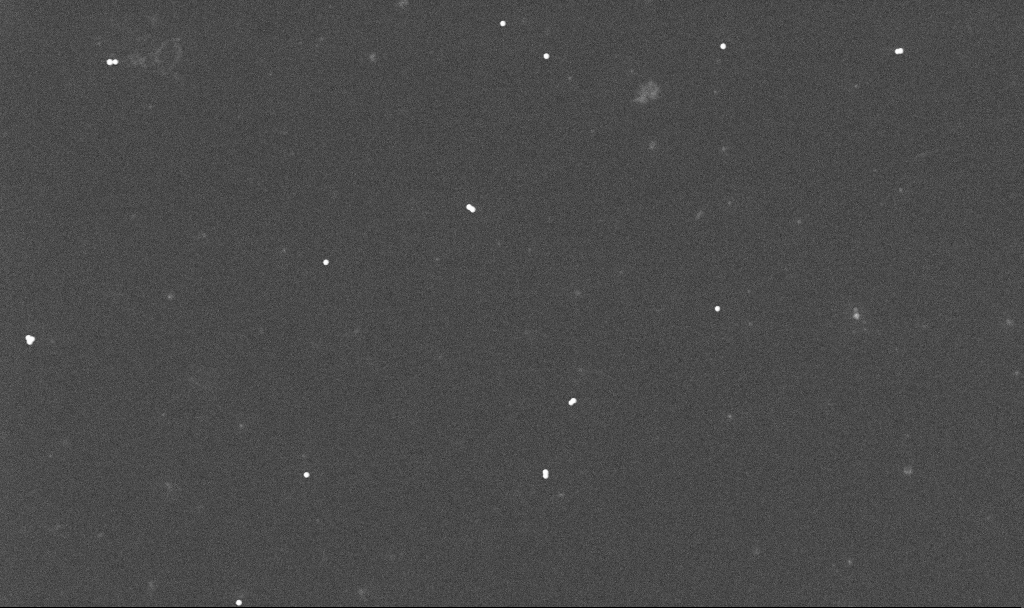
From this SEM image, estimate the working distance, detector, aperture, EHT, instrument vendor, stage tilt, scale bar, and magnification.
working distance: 3.4 mm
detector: InLens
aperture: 30 µm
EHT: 10 kV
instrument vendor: Zeiss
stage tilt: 0°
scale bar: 1000 nm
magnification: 22.42 K X